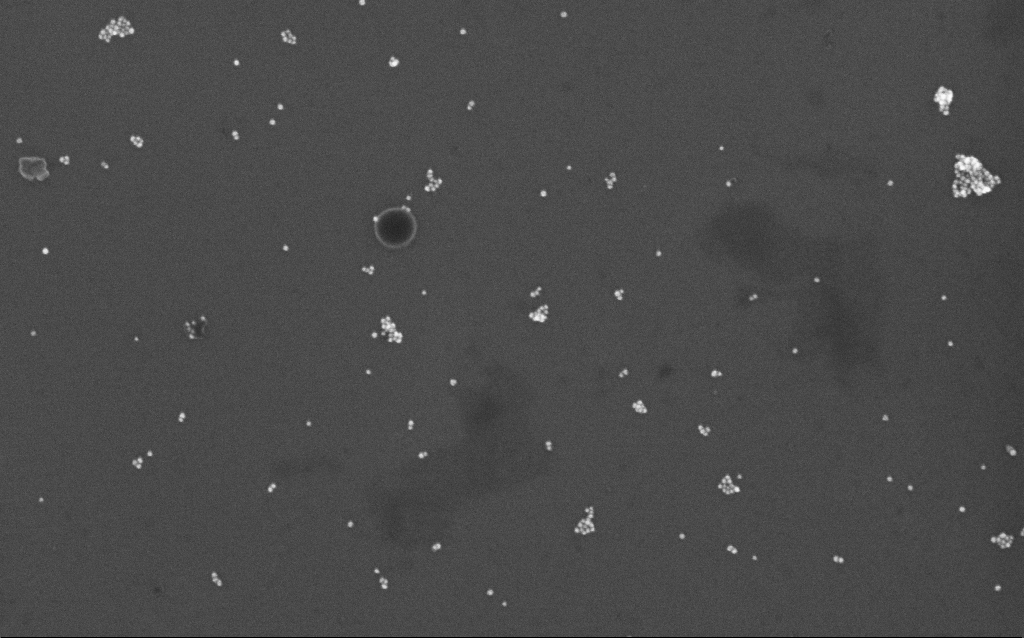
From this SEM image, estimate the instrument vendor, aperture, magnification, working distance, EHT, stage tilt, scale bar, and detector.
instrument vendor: Zeiss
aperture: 30 µm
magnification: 100 K X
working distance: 7 mm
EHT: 10 kV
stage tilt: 0°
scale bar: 200 nm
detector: InLens